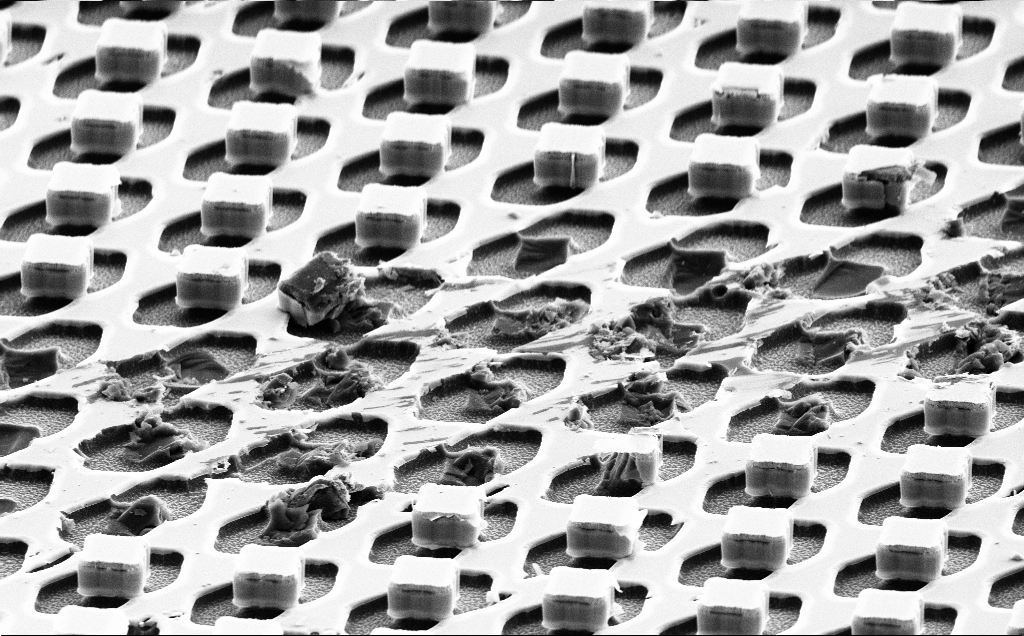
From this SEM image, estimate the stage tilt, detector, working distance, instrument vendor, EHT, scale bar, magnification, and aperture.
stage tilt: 61.7°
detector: SE2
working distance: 12 mm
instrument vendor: Zeiss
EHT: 10 kV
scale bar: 10000 nm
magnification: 2.87 K X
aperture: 30 µm